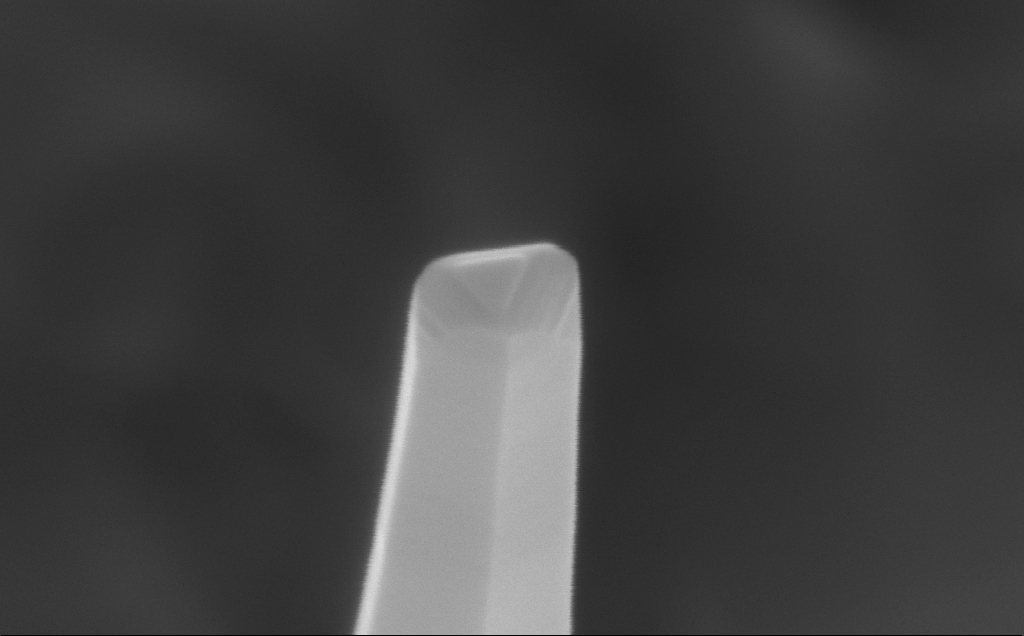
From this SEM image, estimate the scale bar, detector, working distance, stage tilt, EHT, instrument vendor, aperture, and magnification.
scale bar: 100 nm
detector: InLens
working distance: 5 mm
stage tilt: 0°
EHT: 10 kV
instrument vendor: Zeiss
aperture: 30 µm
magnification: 375.31 K X